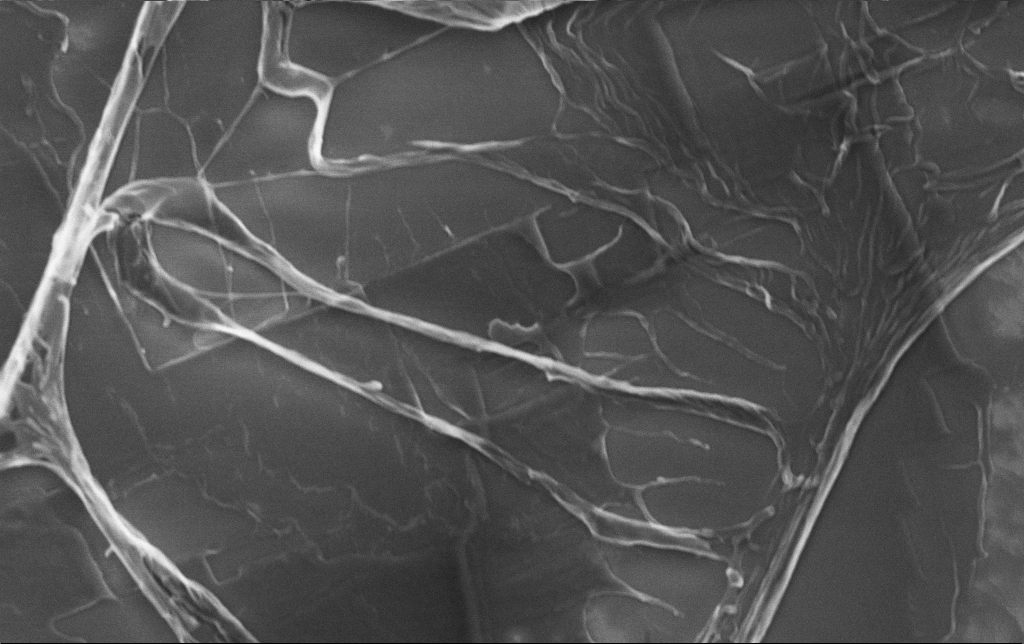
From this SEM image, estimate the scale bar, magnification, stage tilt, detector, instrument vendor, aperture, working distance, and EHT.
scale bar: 2000 nm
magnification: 13.17 K X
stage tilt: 0°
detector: InLens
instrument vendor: Zeiss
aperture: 30 µm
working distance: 3.1 mm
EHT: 5 kV